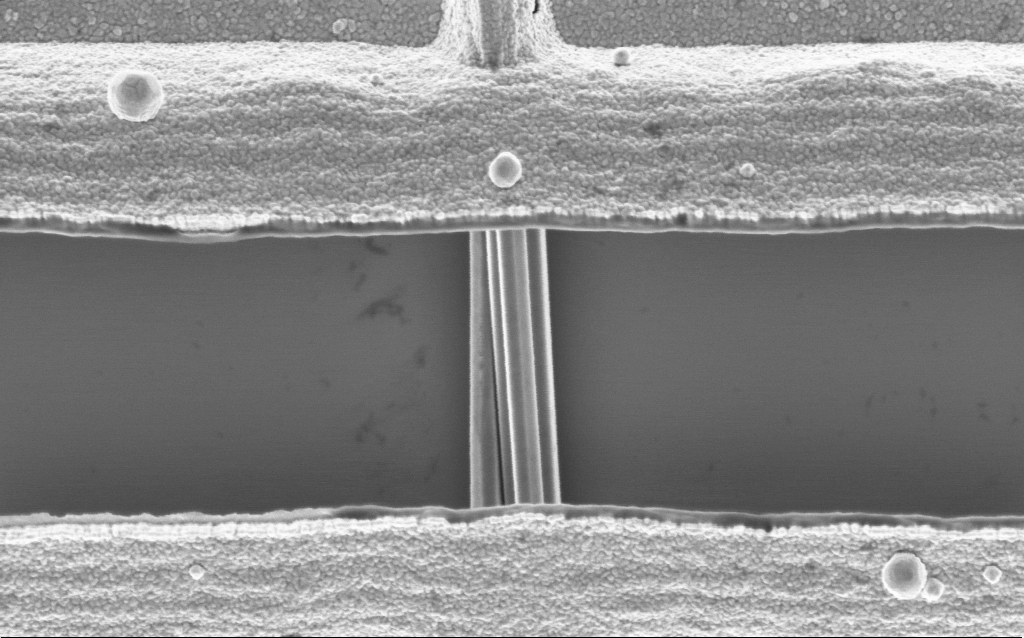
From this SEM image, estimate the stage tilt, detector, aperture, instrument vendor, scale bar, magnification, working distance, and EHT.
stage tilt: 0°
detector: InLens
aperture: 30 µm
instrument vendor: Zeiss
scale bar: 1000 nm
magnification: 63.69 K X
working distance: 7.7 mm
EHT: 2 kV